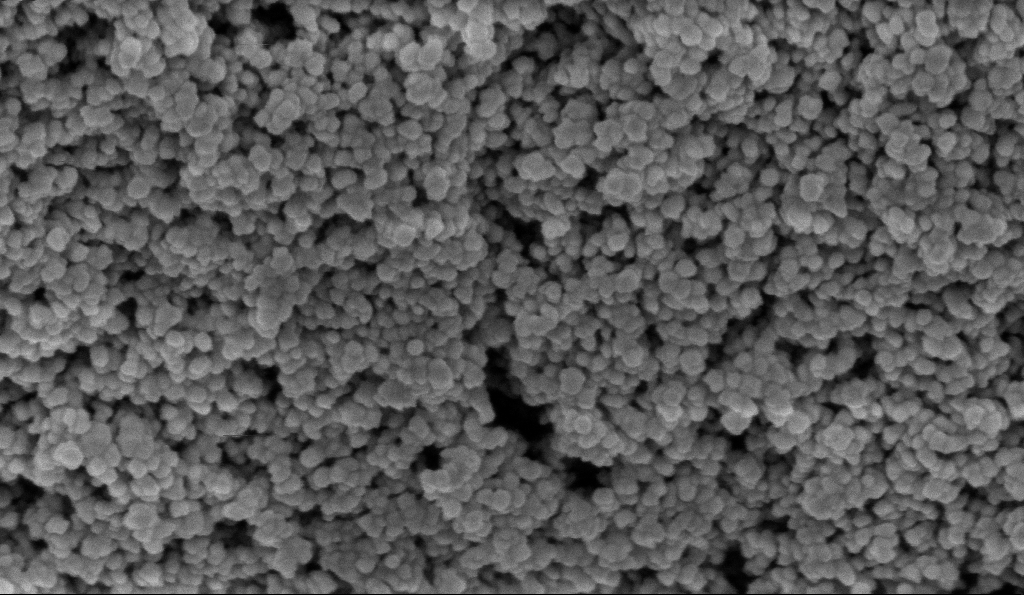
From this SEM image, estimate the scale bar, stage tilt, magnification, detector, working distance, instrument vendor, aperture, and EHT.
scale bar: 200 nm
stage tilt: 0°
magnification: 152.94 K X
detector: InLens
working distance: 5.9 mm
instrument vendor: Zeiss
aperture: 30 µm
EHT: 5 kV